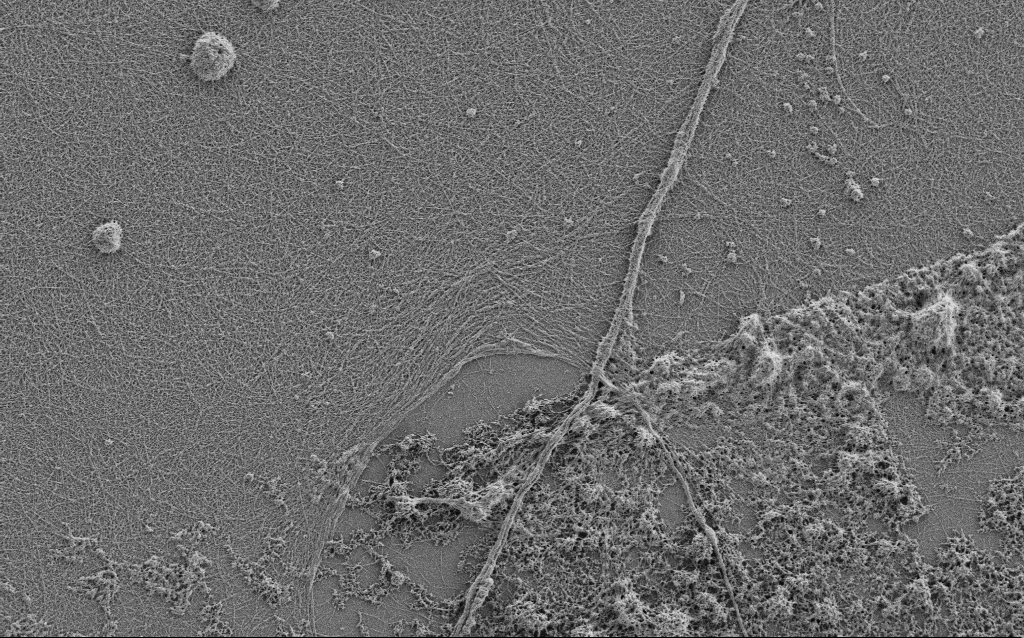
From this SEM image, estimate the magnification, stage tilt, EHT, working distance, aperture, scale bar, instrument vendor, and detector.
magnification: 7.5 K X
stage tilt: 0°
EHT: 0.9 kV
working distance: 4 mm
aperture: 30 µm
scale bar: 2000 nm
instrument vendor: Zeiss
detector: SE2